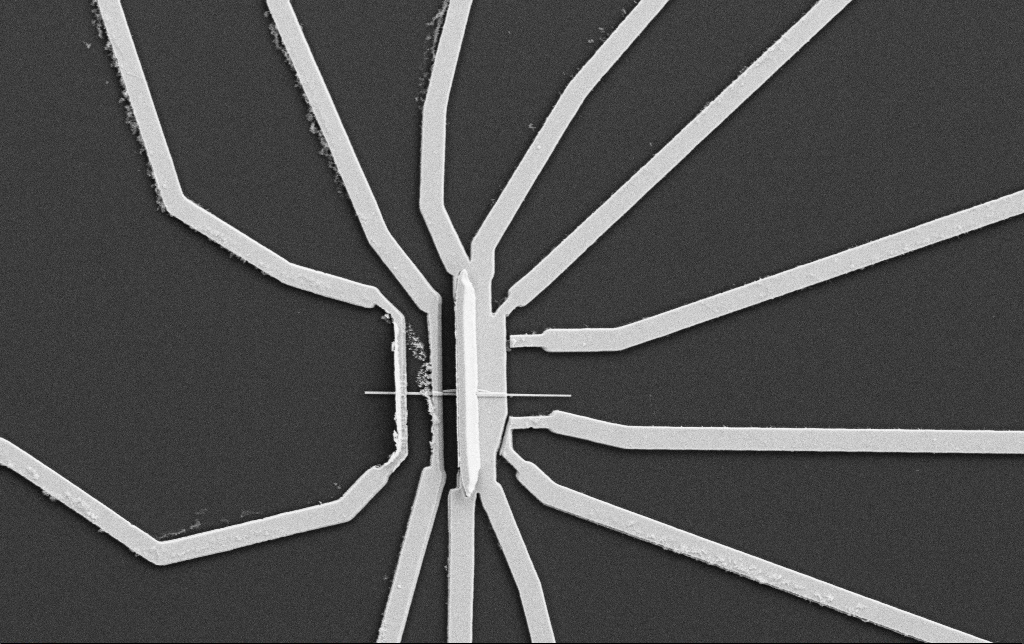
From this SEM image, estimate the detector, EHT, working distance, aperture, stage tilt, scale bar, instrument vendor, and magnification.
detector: SE2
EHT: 5 kV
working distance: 10.7 mm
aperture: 30 µm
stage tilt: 0°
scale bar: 2000 nm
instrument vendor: Zeiss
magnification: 10 K X